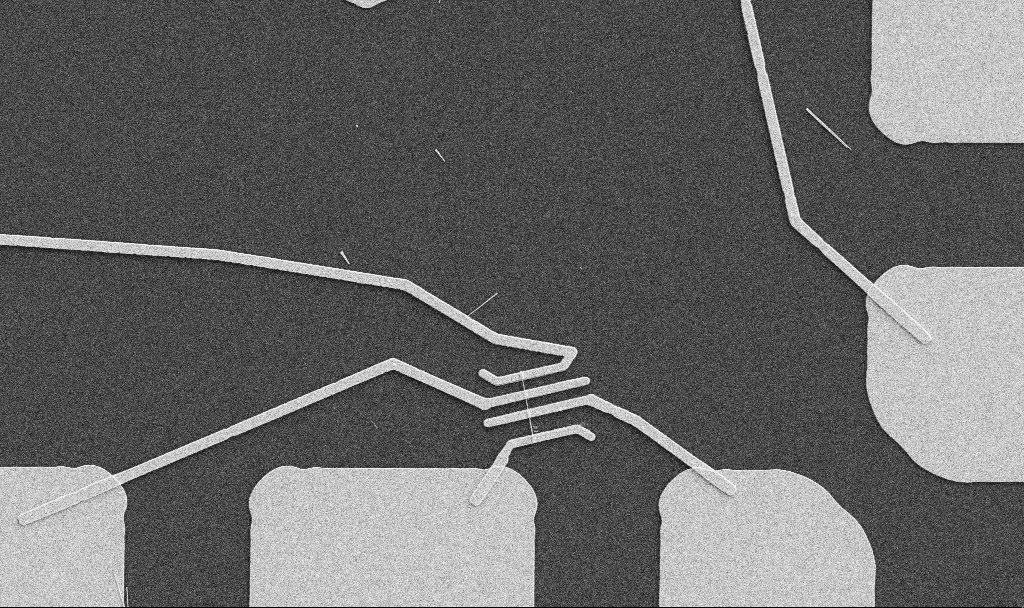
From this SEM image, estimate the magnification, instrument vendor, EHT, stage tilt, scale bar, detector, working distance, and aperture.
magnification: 5 K X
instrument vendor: Zeiss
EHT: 5 kV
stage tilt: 0°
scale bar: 10000 nm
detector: SE2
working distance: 10.7 mm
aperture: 30 µm